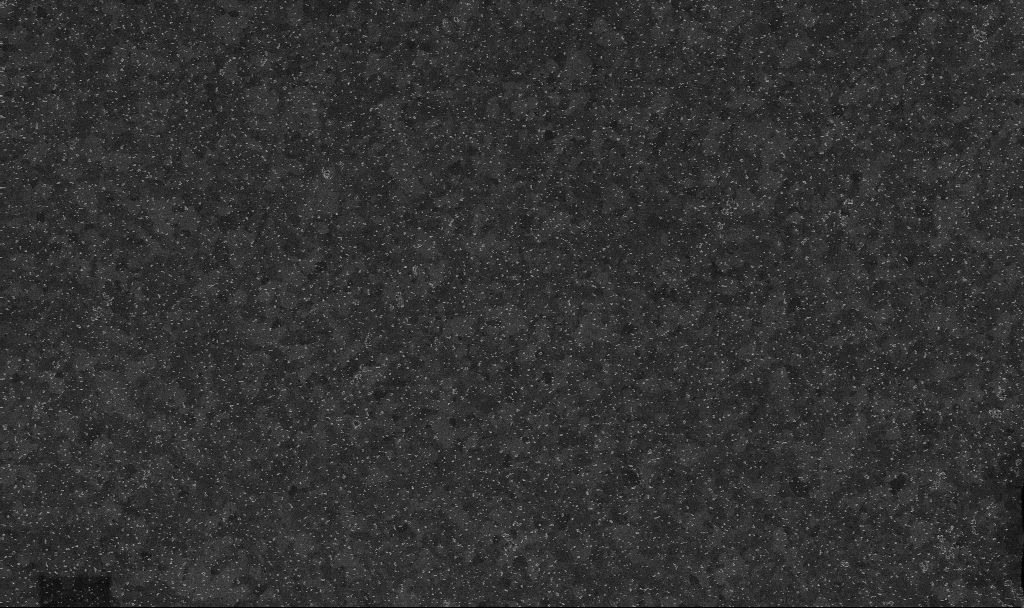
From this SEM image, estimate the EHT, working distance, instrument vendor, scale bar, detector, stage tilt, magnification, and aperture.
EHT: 10 kV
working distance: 3.4 mm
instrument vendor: Zeiss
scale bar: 1000 nm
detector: InLens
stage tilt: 0°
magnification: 20 K X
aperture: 30 µm